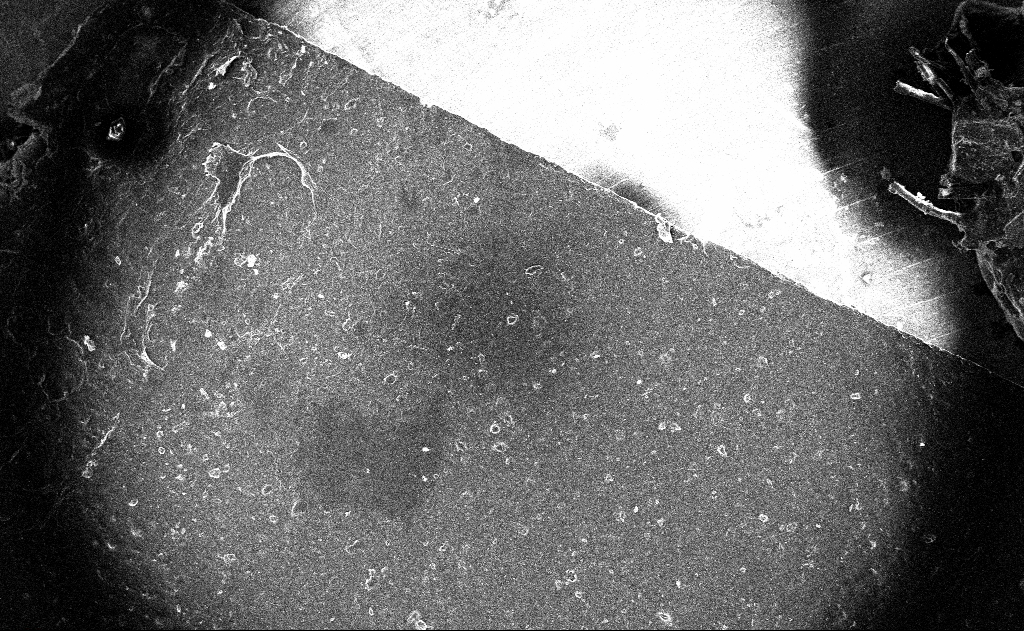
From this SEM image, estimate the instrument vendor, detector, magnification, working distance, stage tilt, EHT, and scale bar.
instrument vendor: Zeiss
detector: InLens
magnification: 0.122 K X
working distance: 3 mm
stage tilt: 0°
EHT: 10 kV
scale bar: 100000 nm